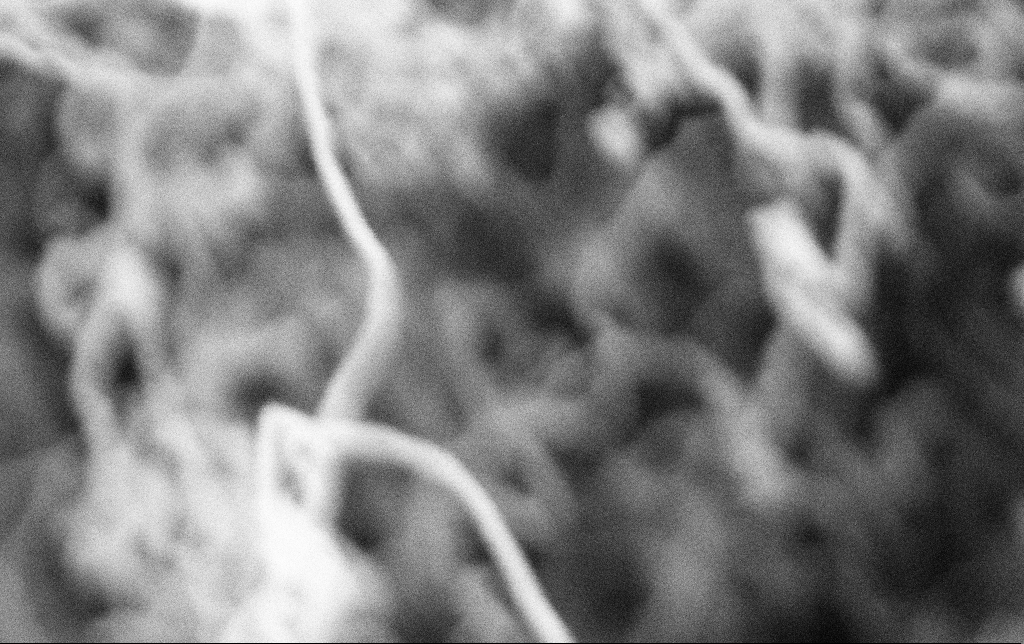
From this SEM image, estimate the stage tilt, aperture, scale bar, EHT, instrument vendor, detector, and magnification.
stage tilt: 0°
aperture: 30 µm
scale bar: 200 nm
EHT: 2 kV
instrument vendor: Zeiss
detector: SE2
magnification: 100 K X